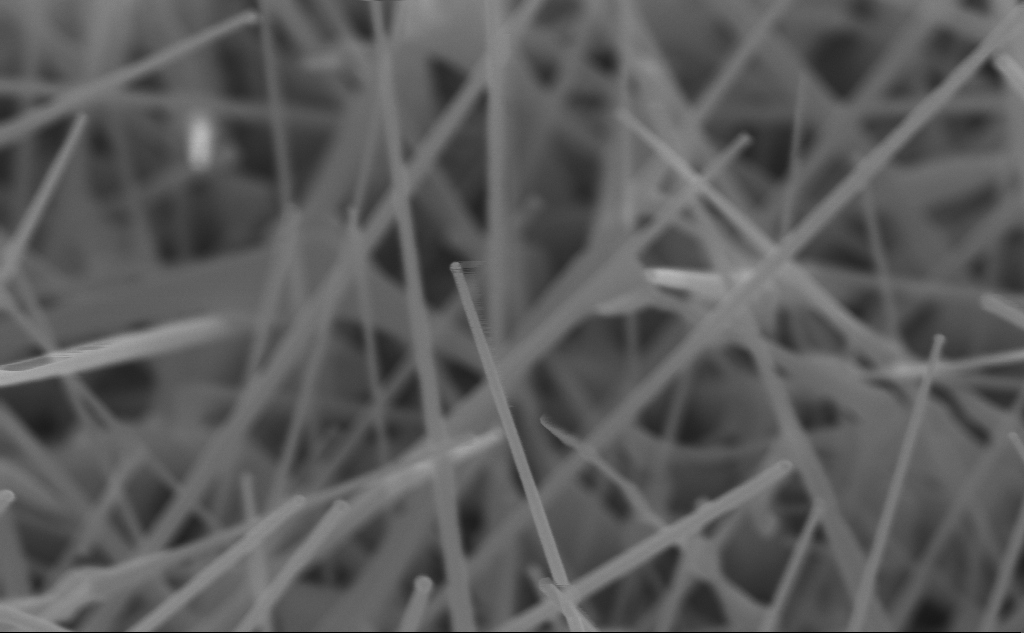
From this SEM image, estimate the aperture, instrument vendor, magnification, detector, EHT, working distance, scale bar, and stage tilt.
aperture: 30 µm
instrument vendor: Zeiss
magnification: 119.72 K X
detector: InLens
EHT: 10 kV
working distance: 5 mm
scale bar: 200 nm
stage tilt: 45°